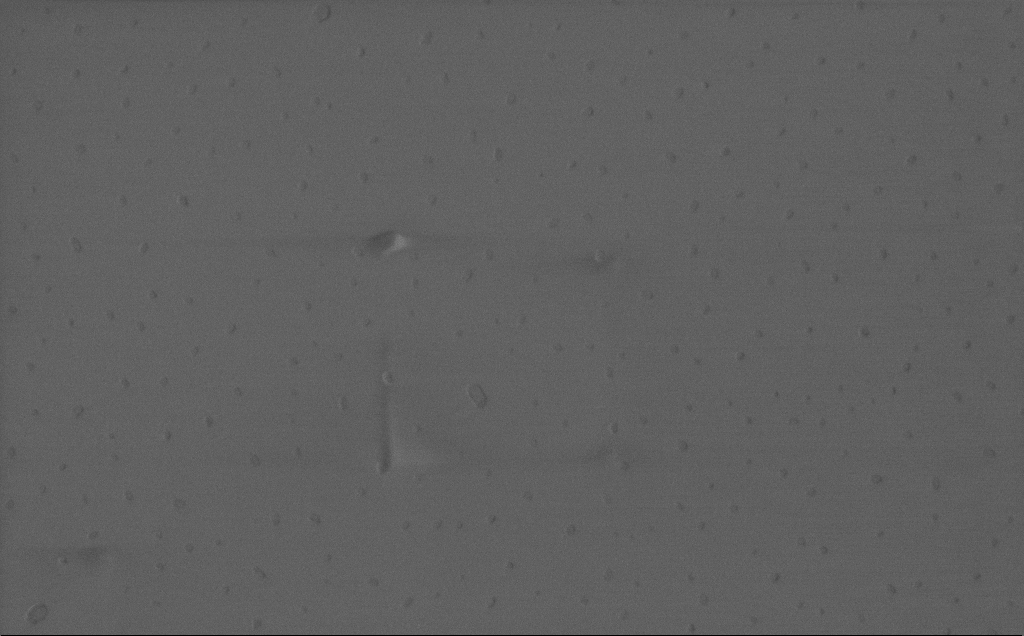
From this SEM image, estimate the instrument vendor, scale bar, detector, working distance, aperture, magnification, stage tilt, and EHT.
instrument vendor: Zeiss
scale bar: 1000 nm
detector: InLens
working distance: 3 mm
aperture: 30 µm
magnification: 45.29 K X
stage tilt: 0°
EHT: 1 kV